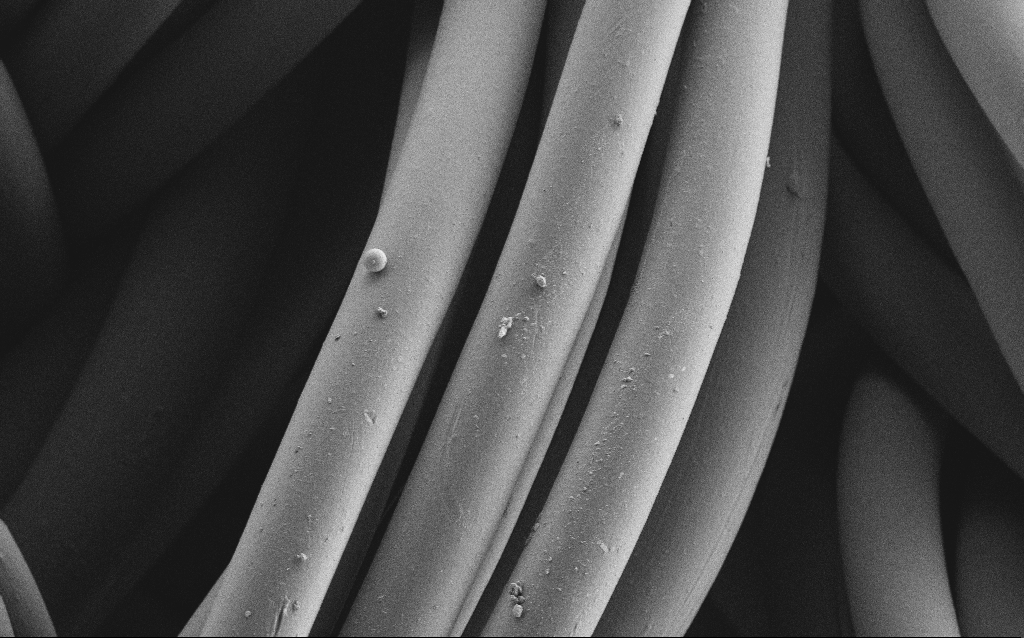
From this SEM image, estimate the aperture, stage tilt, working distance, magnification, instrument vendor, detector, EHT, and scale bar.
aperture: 30 µm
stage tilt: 0°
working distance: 4 mm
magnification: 1.58 K X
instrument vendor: Zeiss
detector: SE2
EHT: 1 kV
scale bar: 20000 nm